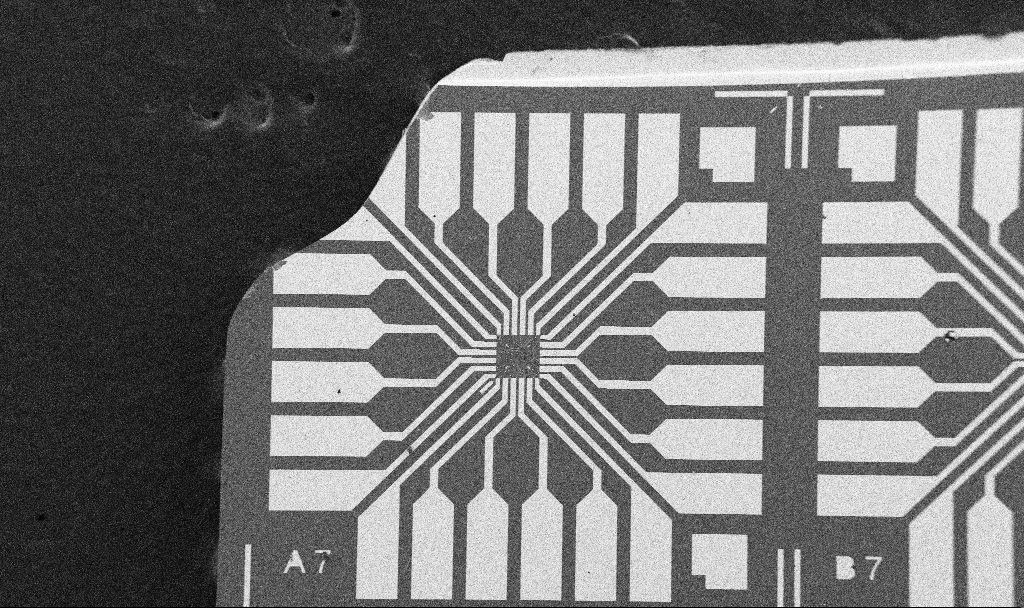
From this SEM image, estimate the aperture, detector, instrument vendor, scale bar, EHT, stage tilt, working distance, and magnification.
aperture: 30 µm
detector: SE2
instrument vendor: Zeiss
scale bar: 200000 nm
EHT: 5 kV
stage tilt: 0°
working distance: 10.7 mm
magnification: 0.1 K X